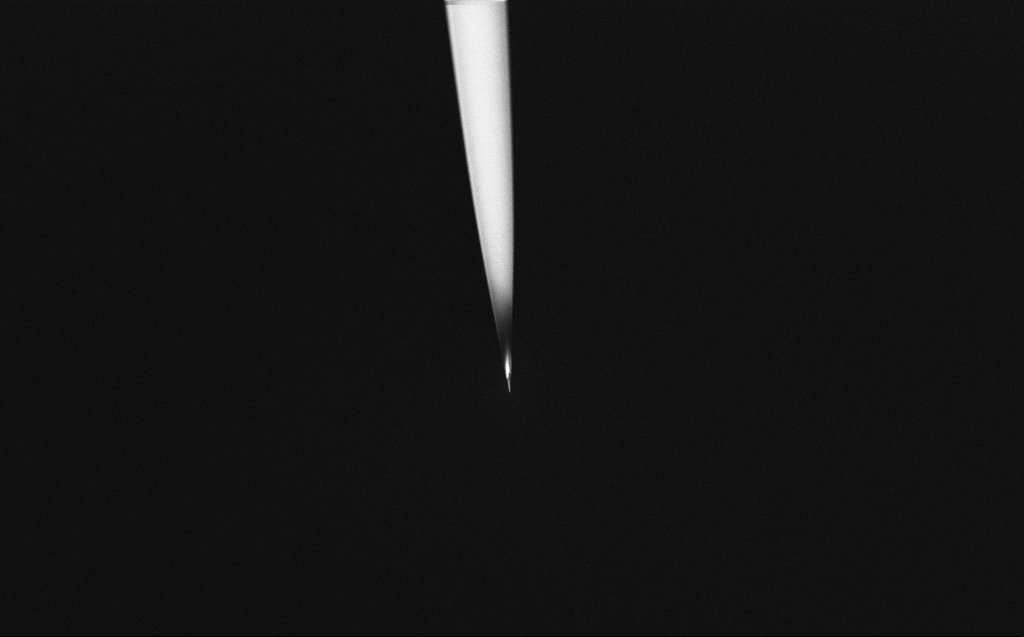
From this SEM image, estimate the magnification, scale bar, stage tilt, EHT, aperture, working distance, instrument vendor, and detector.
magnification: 1 K X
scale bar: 20000 nm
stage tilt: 45°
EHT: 2 kV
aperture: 30 µm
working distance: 6 mm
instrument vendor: Zeiss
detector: InLens